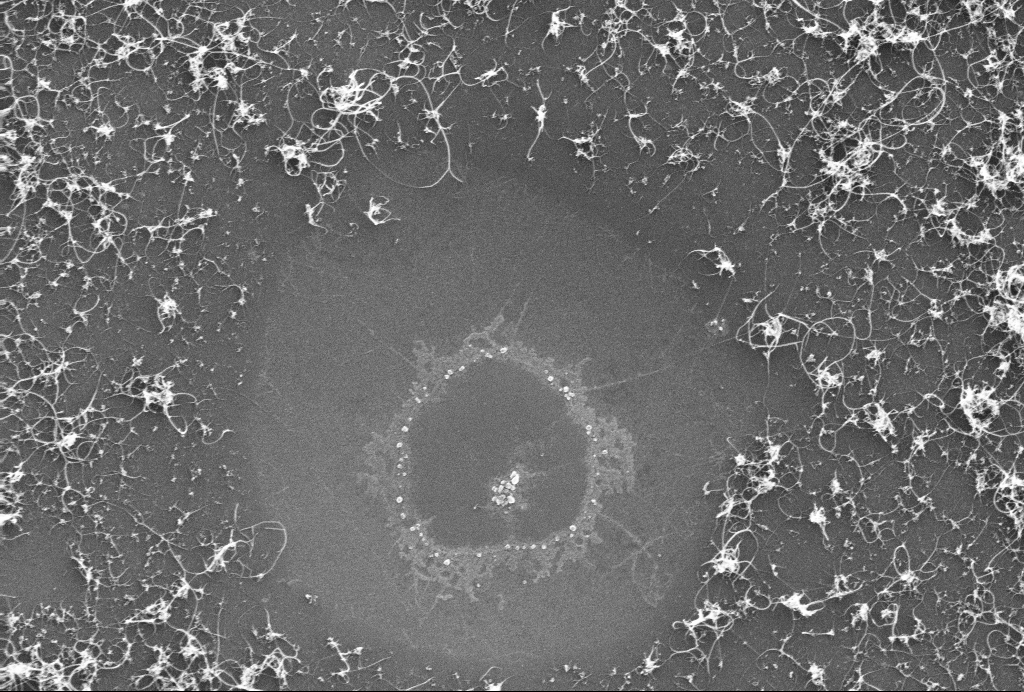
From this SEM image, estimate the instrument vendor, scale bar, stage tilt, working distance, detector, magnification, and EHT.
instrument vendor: Zeiss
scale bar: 1000 nm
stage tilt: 0°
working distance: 3.1 mm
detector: InLens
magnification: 54.26 K X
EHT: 10 kV